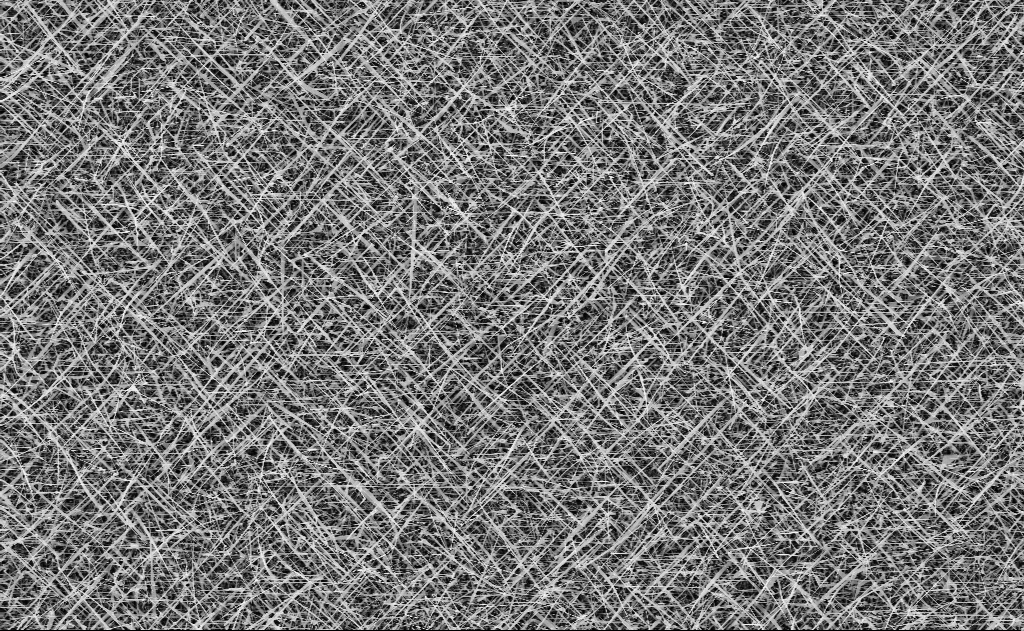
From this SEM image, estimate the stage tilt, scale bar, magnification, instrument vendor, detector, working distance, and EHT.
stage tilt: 0°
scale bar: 10000 nm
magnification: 5 K X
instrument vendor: Zeiss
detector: InLens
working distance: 11 mm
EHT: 10 kV